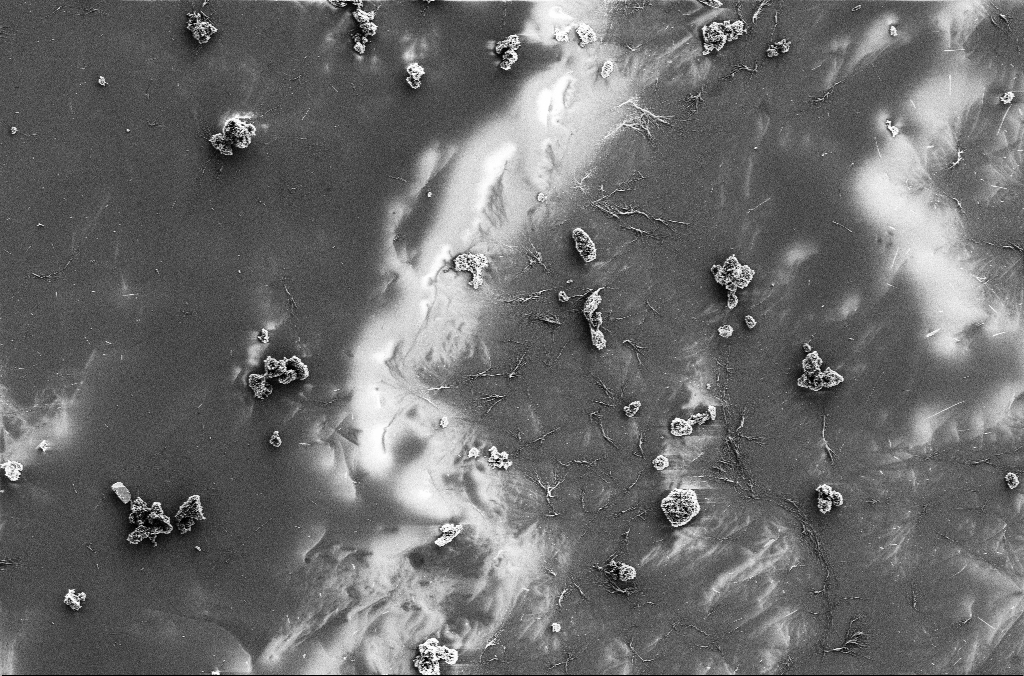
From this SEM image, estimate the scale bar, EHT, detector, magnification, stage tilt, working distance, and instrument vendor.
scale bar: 10000 nm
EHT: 3 kV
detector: SE2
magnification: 2.5 K X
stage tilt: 0°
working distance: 5.1 mm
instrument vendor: Zeiss